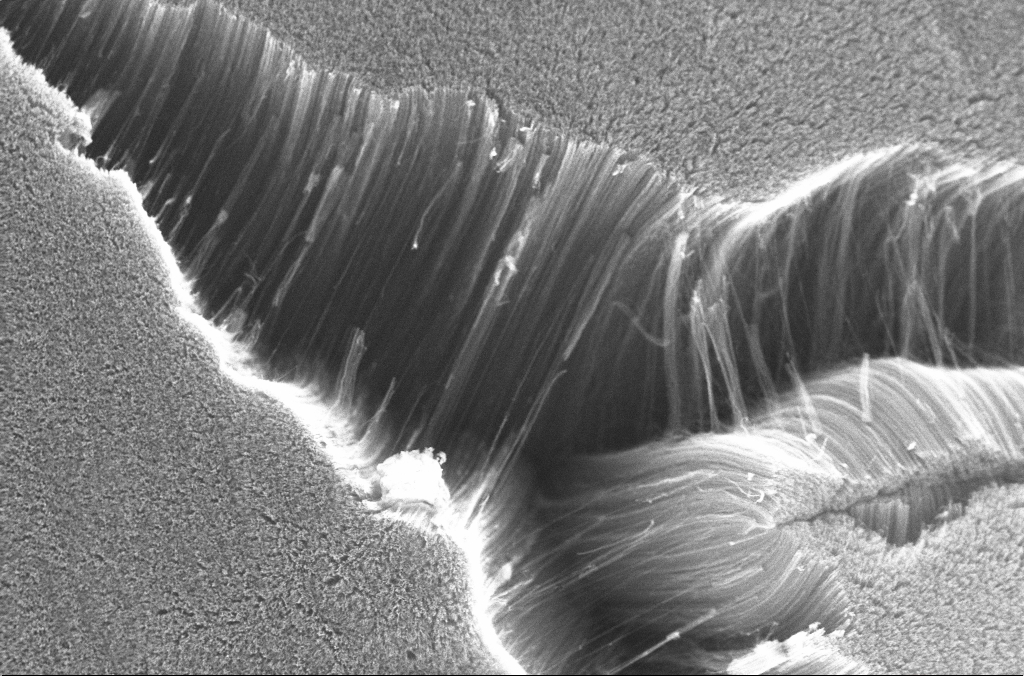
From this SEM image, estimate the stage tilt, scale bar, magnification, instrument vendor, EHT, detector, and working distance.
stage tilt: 0°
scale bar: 20000 nm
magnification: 1 K X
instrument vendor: Zeiss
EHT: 20 kV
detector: InLens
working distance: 3.9 mm